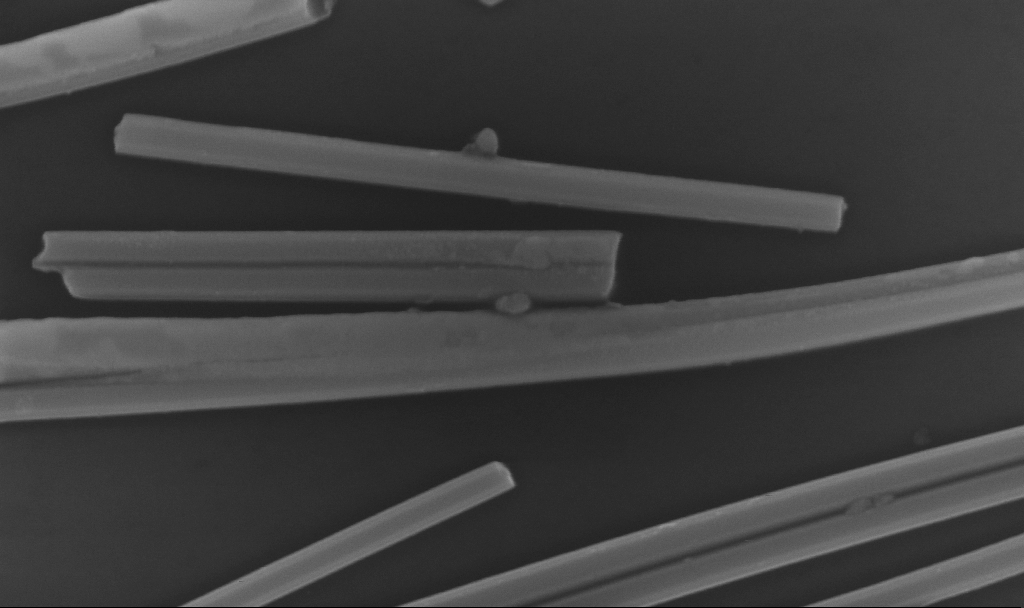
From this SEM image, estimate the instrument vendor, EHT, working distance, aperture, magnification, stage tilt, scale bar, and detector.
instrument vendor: Zeiss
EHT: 10 kV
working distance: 6.7 mm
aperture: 30 µm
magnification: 146.49 K X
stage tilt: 0°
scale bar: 200 nm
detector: InLens